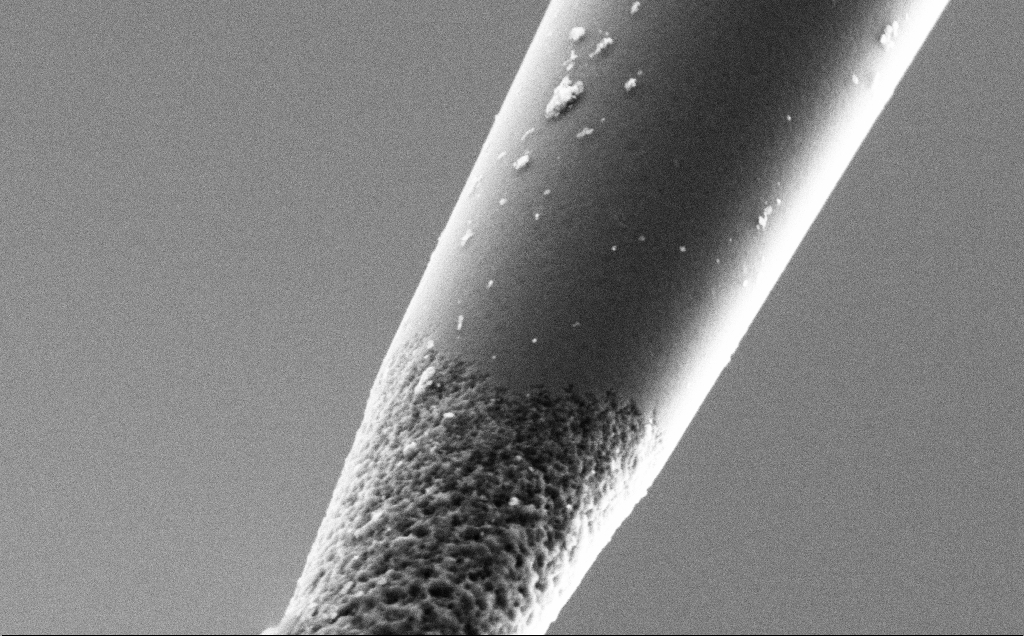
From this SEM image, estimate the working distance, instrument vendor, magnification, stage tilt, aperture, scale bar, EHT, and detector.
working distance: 7.7 mm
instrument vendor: Zeiss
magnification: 50 K X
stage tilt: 45°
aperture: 30 µm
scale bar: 1000 nm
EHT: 3 kV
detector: SE2